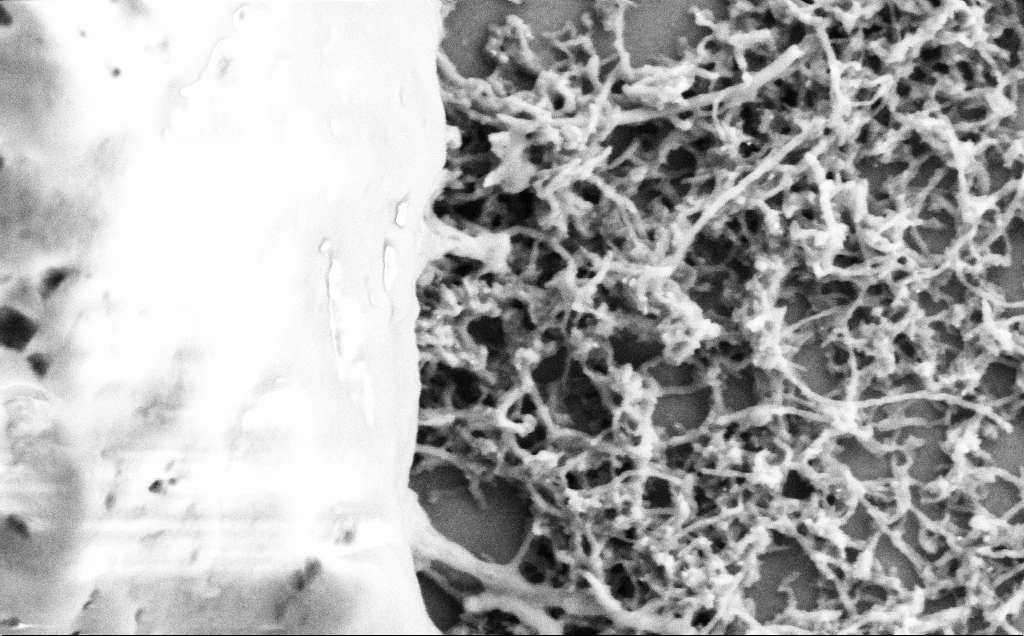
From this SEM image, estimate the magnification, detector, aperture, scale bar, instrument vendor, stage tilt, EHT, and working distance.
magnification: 75 K X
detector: SE2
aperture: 30 µm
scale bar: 200 nm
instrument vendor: Zeiss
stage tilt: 0°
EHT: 2 kV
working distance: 7.1 mm